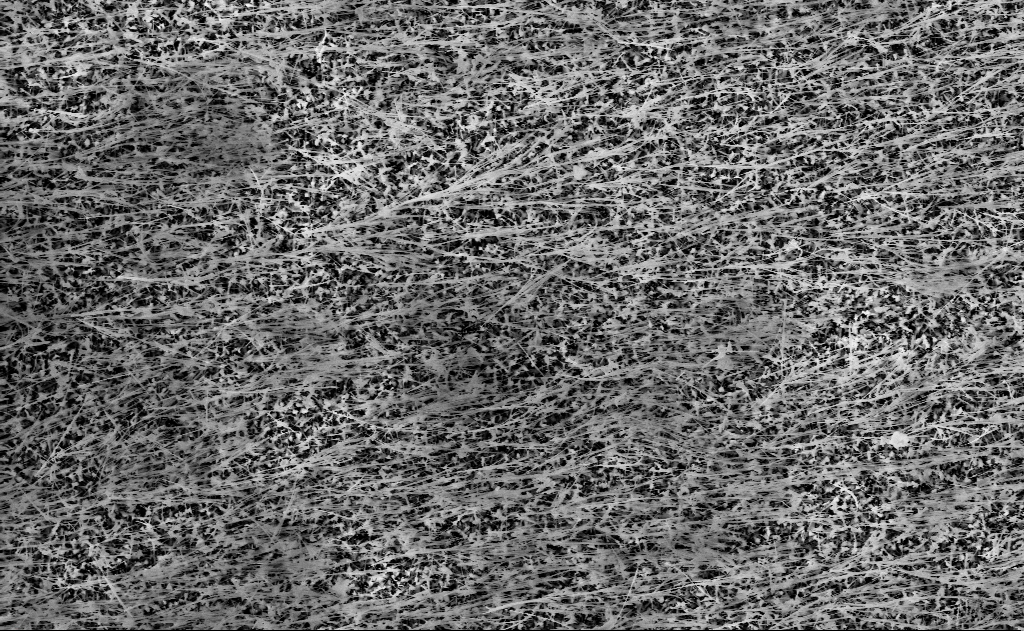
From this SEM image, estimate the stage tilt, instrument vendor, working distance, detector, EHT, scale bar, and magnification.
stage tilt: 0°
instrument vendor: Zeiss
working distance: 15 mm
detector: InLens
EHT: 10 kV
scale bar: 2000 nm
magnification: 10 K X